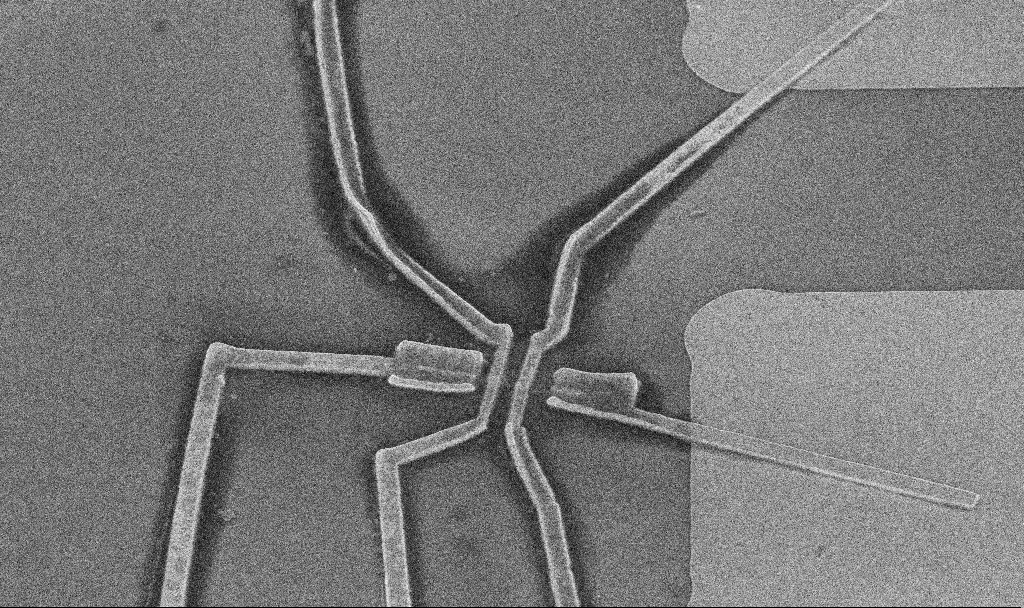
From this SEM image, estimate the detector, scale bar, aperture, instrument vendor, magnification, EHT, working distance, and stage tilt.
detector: InLens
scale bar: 2000 nm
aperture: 30 µm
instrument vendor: Zeiss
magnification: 10 K X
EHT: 5 kV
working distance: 12.4 mm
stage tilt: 45°